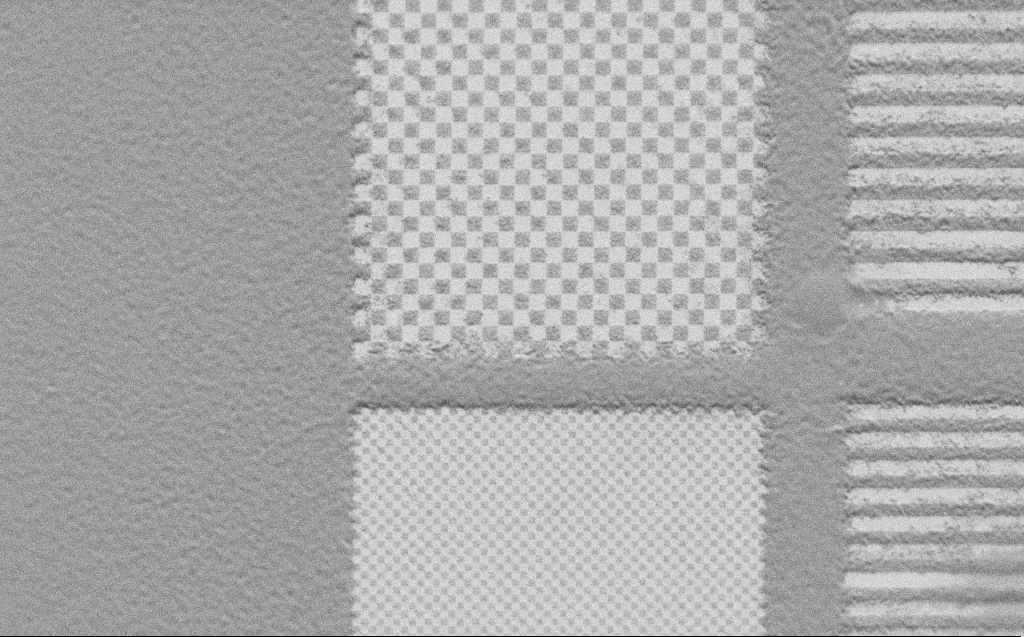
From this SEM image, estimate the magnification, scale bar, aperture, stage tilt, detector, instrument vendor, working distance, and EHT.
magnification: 5.92 K X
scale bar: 10000 nm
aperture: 30 µm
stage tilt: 45°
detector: SE2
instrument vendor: Zeiss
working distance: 5 mm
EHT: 1 kV